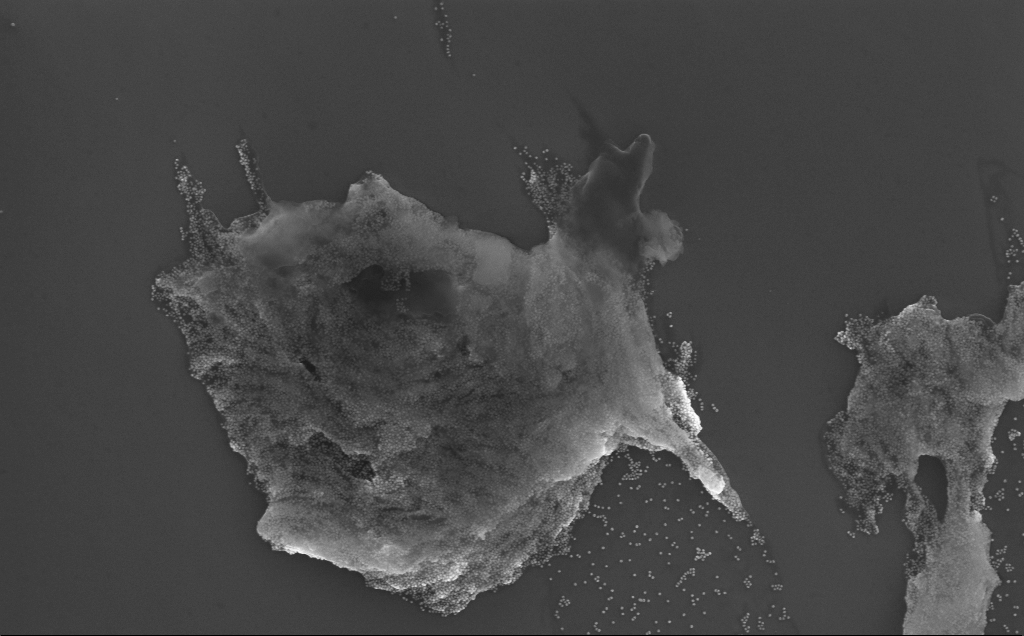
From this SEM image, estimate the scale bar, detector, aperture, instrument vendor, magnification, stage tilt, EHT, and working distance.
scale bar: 1000 nm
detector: InLens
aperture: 30 µm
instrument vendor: Zeiss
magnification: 57.64 K X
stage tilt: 0°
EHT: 10 kV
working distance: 3 mm